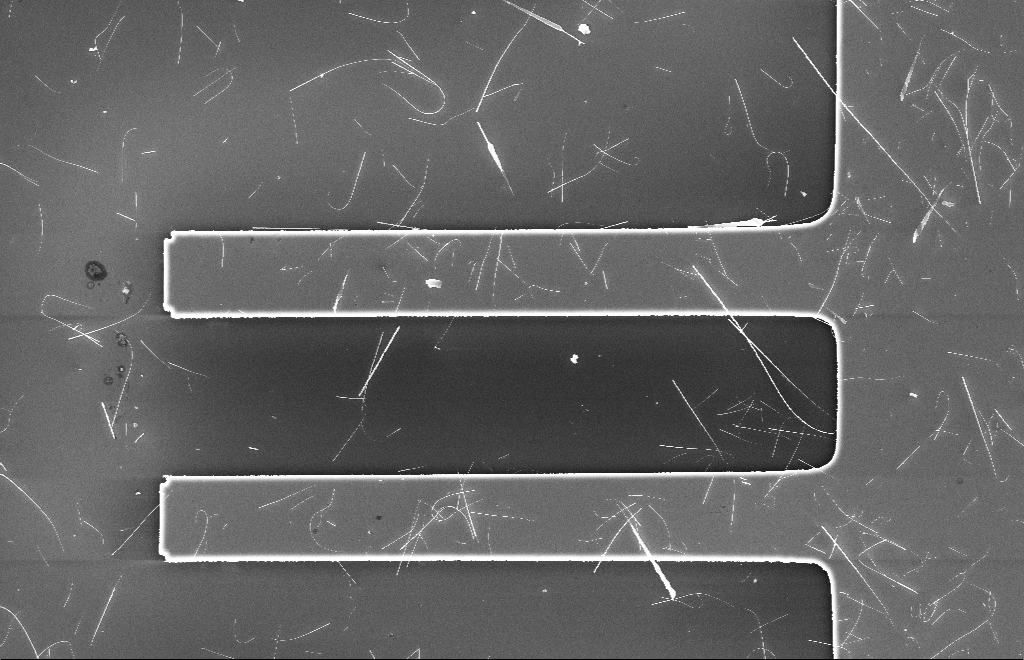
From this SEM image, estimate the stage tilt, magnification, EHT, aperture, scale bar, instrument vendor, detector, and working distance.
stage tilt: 0°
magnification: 2.5 K X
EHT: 10 kV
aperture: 20 µm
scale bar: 10000 nm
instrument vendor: Zeiss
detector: InLens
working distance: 6 mm